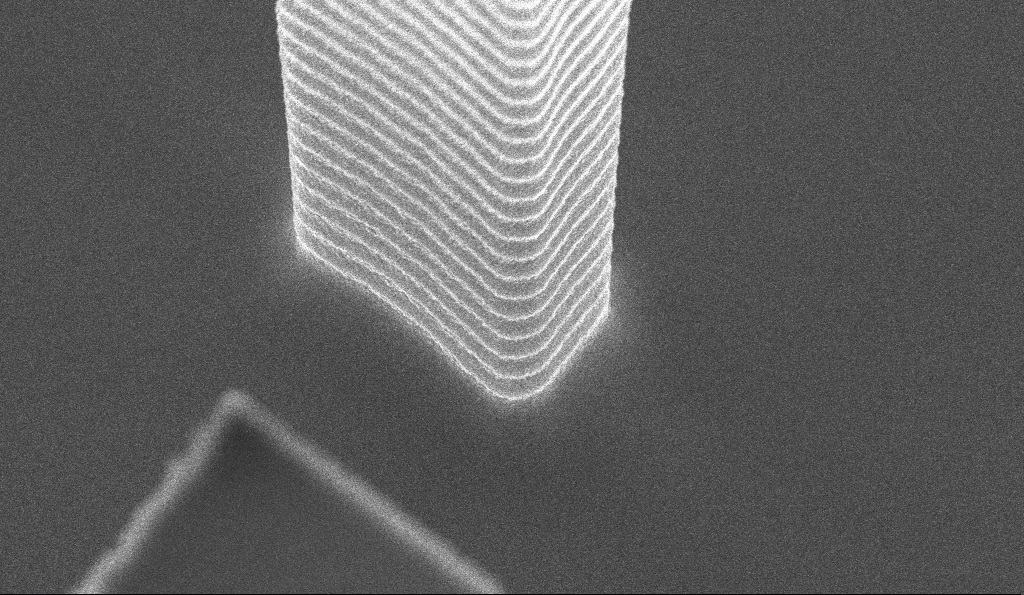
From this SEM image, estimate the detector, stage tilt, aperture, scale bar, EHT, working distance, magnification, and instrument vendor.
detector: InLens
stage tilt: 30°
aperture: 30 µm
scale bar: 2000 nm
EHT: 5 kV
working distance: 5.3 mm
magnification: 33.57 K X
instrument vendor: Zeiss